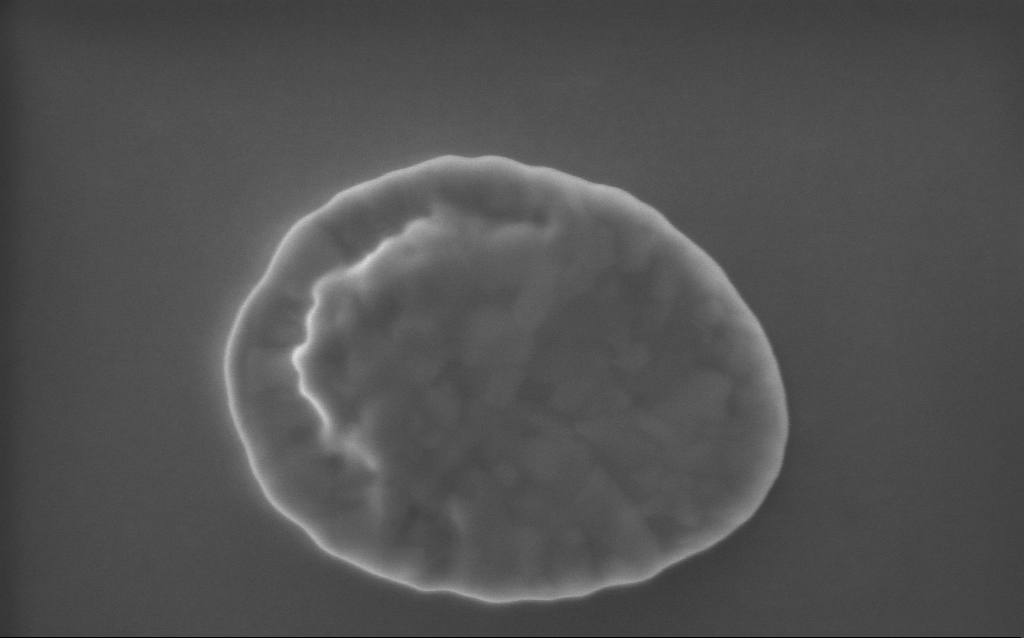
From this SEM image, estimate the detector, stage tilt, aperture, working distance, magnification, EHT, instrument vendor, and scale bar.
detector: InLens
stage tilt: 0°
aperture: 30 µm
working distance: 2 mm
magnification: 100.82 K X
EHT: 5 kV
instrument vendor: Zeiss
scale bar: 200 nm